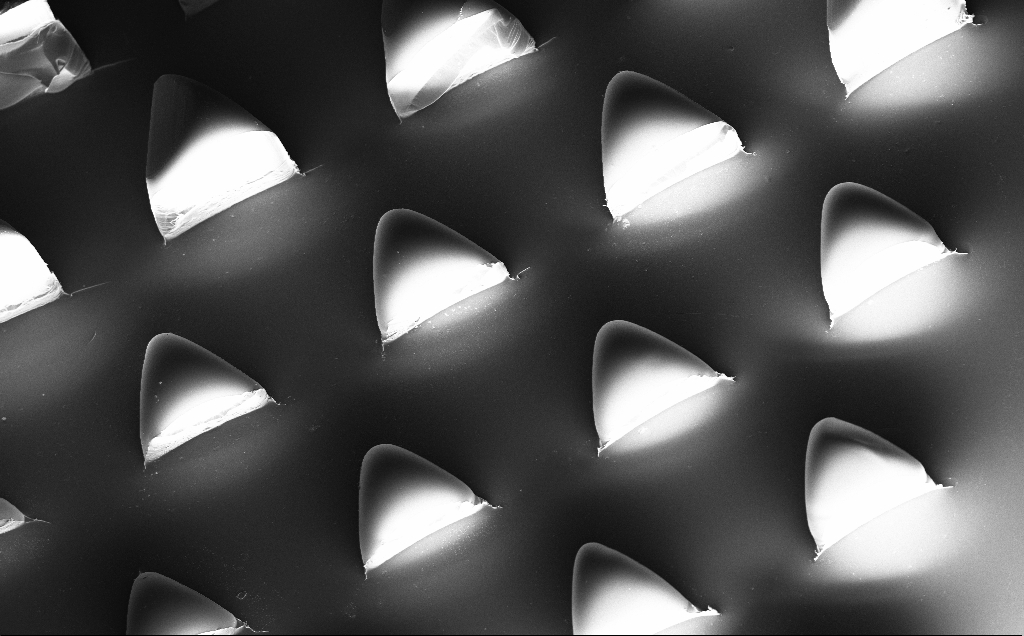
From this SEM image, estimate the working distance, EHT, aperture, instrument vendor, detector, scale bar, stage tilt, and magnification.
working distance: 10 mm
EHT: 10 kV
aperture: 30 µm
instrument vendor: Zeiss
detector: InLens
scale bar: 100000 nm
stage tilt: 20°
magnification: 0.212 K X